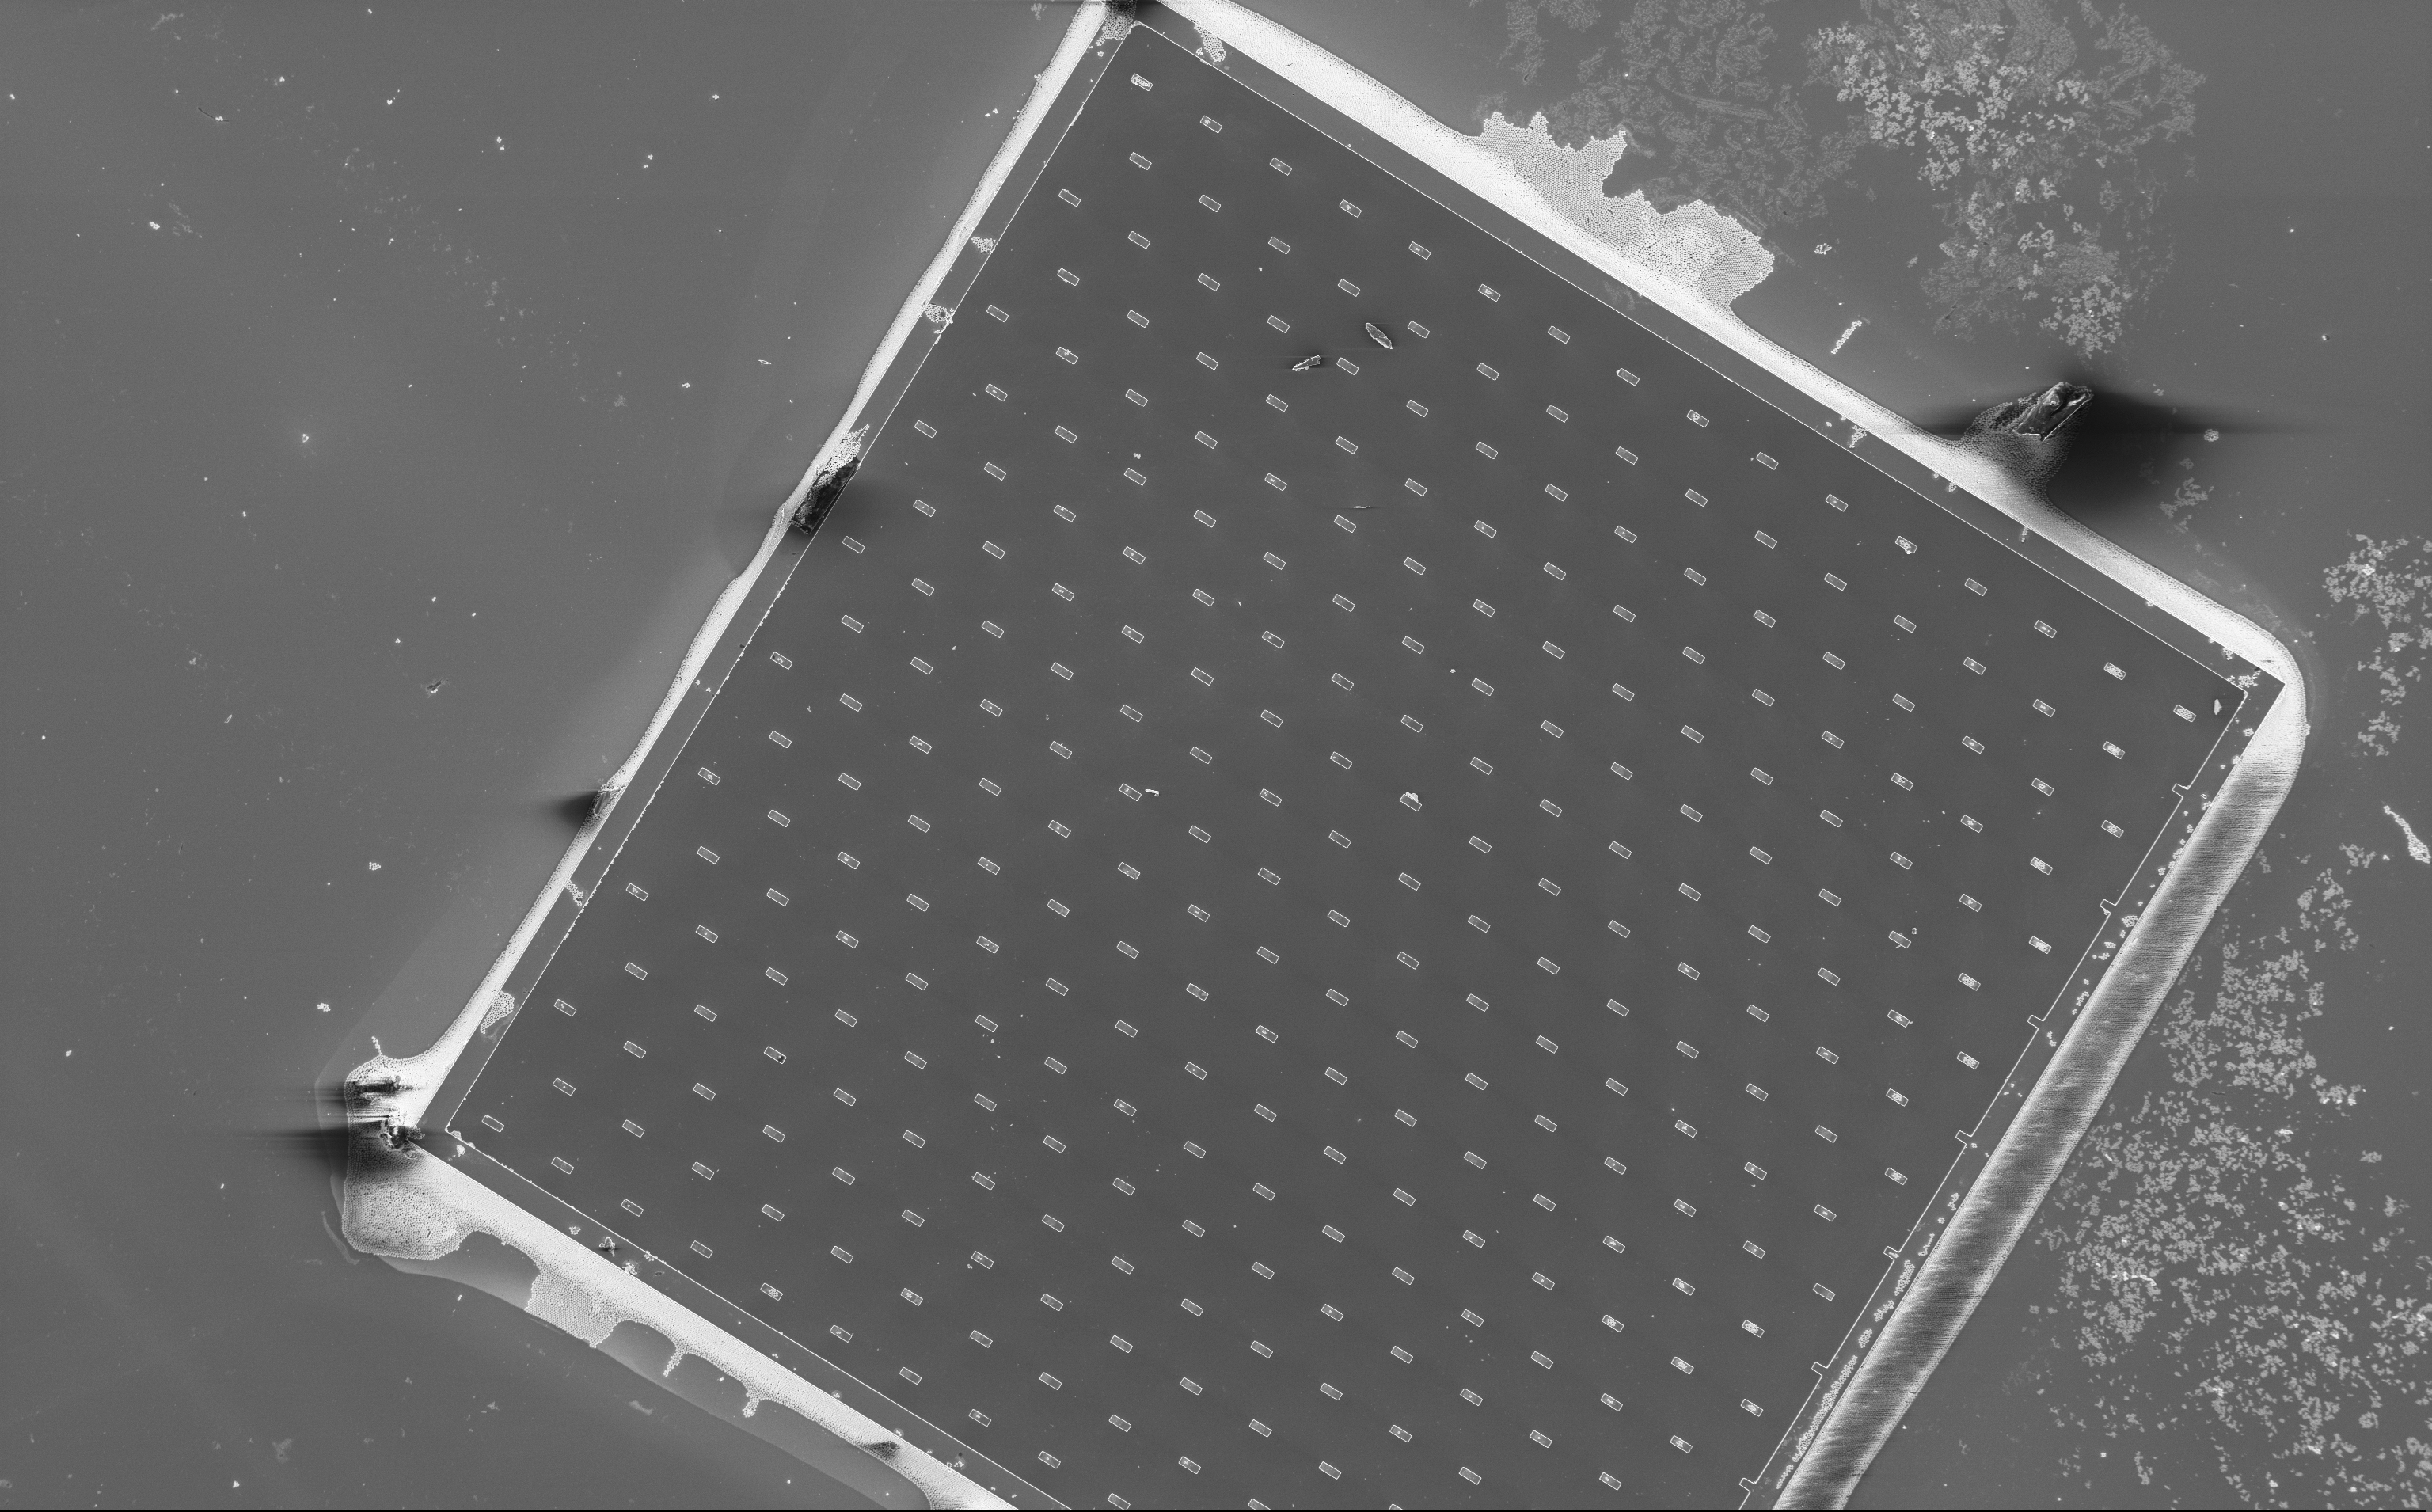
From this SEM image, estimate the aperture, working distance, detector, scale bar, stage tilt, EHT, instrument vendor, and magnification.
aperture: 30 µm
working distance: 8 mm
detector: InLens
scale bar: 100000 nm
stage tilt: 0°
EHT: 5 kV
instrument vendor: Zeiss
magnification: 0.444 K X